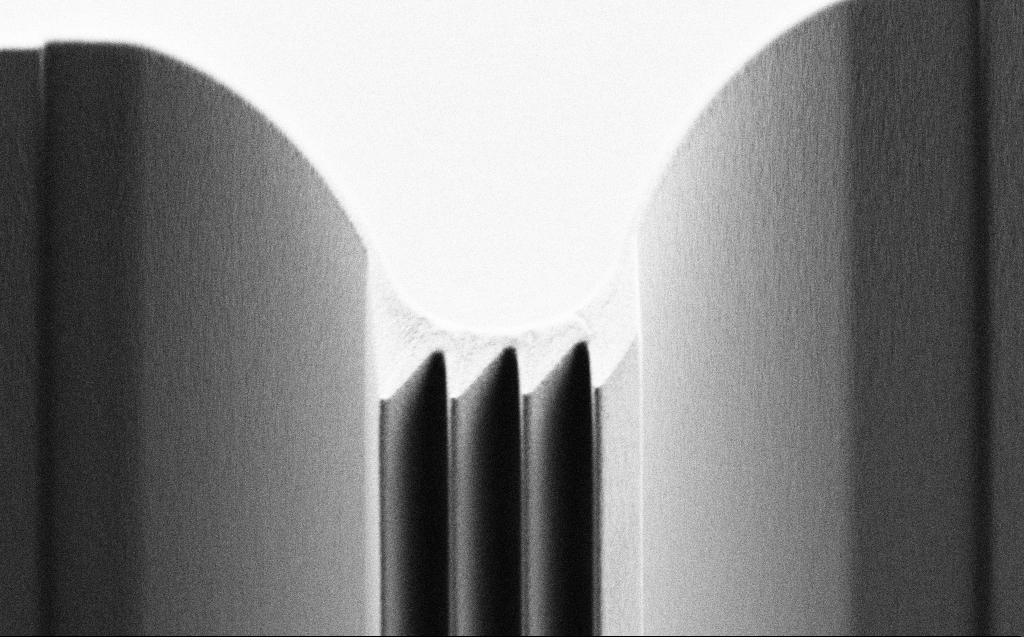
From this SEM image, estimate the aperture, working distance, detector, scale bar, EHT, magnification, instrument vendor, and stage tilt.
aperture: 30 µm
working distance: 4 mm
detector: SE2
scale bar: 20000 nm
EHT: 0.9 kV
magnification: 2.35 K X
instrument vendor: Zeiss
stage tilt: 45°